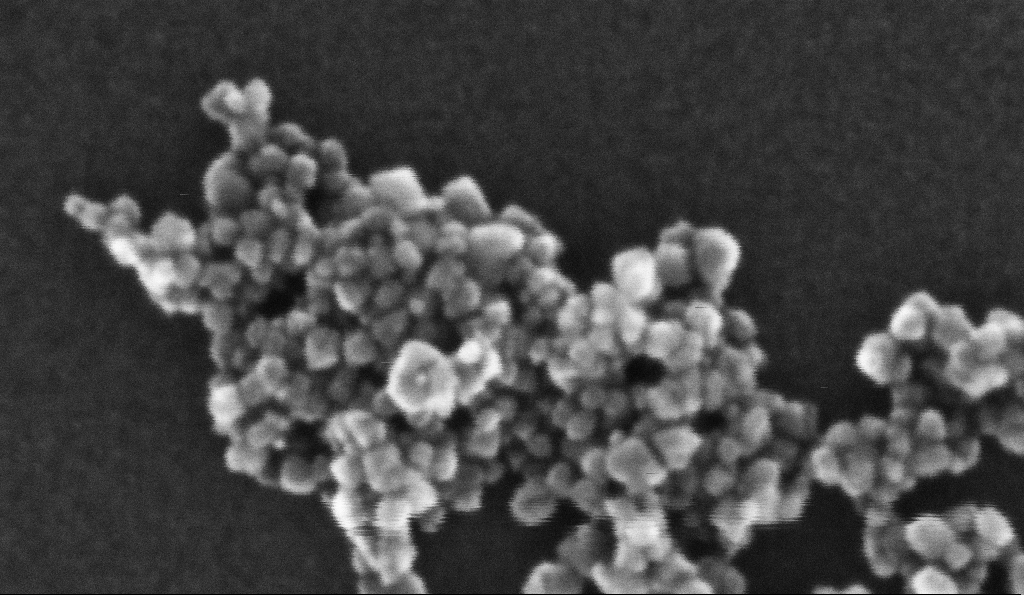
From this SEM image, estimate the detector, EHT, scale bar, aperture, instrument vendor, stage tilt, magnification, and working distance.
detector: InLens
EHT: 10 kV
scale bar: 20 nm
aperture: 30 µm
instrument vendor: Zeiss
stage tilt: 0°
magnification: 901.21 K X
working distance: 5.2 mm